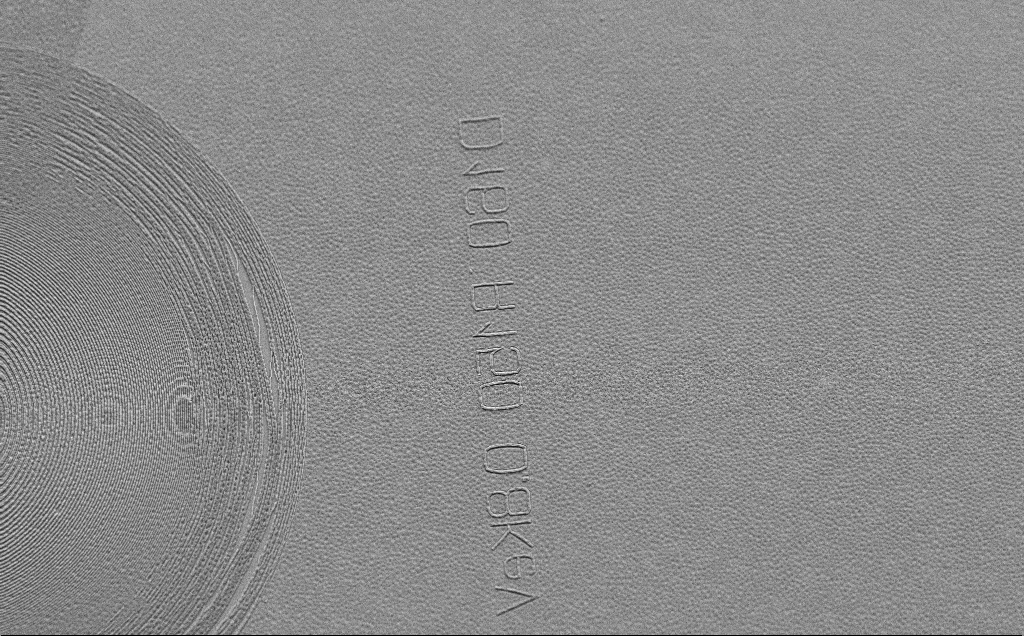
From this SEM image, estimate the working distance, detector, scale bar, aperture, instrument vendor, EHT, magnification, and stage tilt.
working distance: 6 mm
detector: SE2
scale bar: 10000 nm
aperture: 30 µm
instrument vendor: Zeiss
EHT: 5 kV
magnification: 1.79 K X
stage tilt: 45°